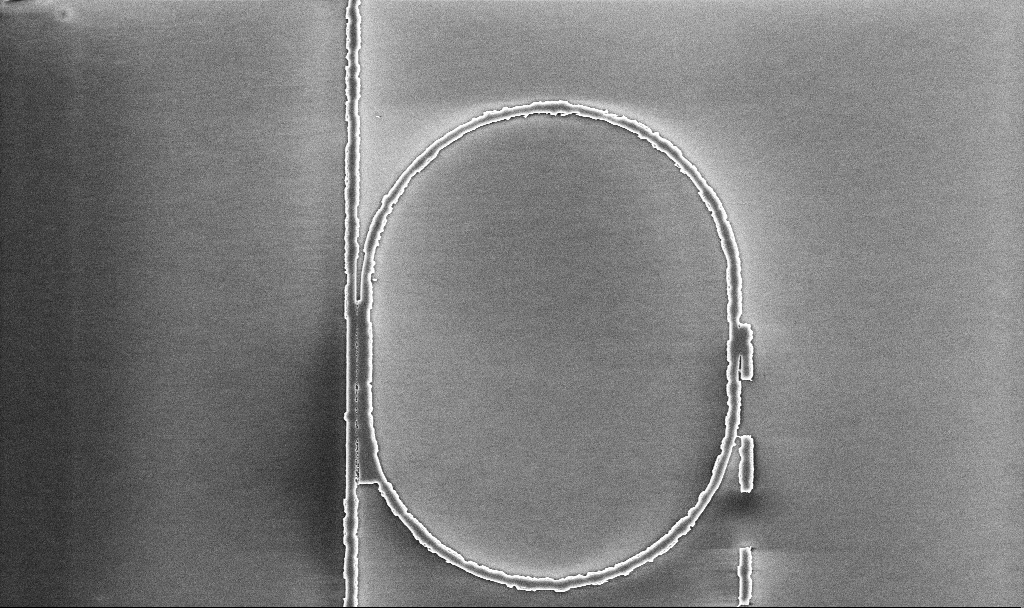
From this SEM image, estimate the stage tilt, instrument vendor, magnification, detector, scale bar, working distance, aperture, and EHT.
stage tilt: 0°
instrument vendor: Zeiss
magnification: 6.98 K X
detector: InLens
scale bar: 10000 nm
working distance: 10.1 mm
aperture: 30 µm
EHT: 5 kV